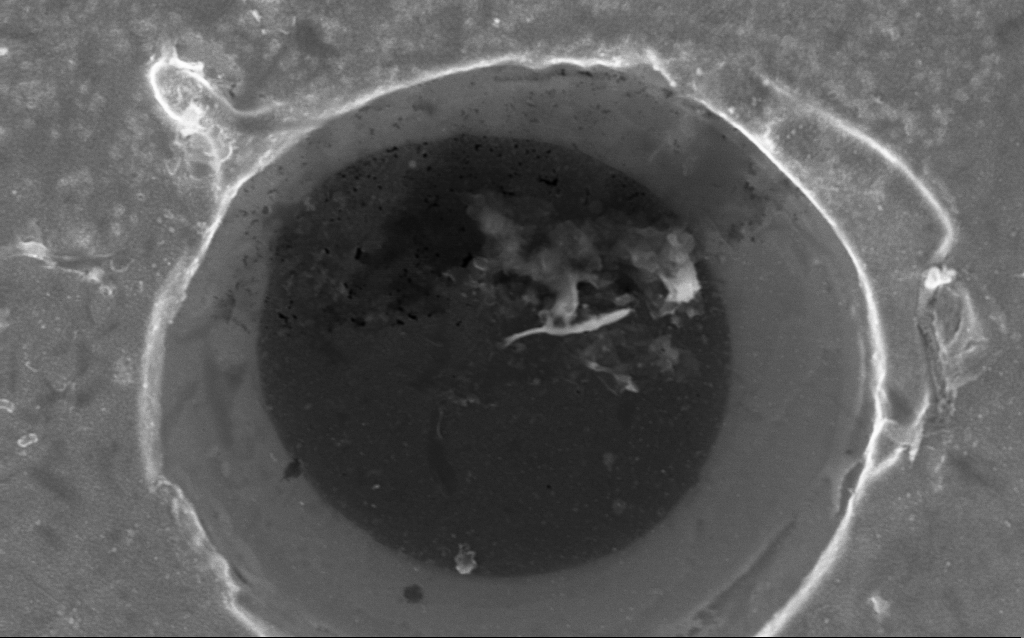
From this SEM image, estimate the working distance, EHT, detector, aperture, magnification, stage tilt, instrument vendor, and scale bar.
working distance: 4.6 mm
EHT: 5 kV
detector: InLens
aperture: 30 µm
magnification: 44.86 K X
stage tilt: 0°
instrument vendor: Zeiss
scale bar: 1000 nm